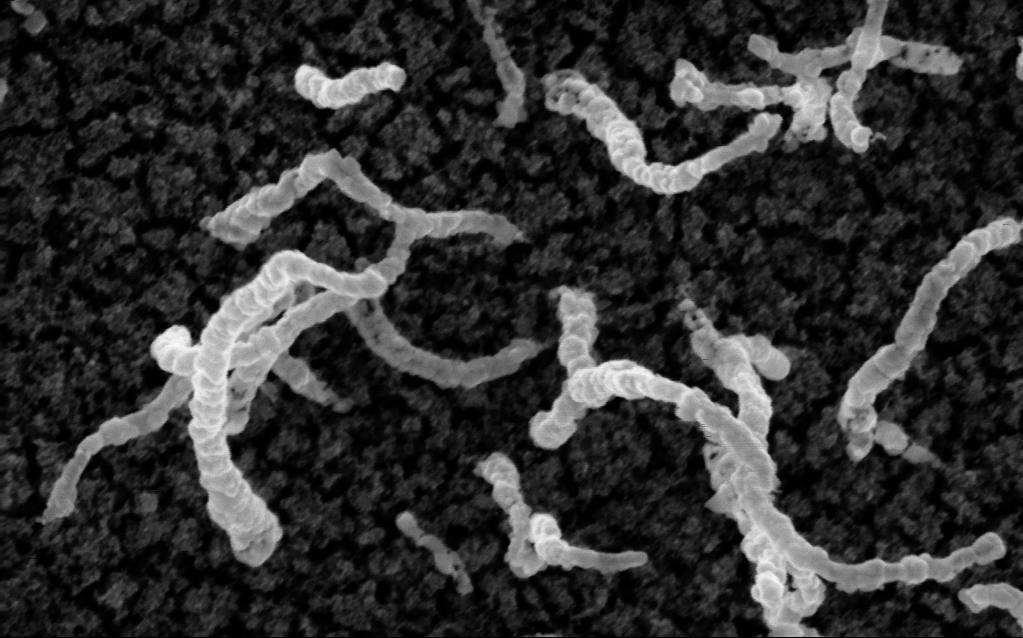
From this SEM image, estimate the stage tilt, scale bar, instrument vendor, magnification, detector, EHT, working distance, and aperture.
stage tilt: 0°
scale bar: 100 nm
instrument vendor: Zeiss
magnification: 200 K X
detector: InLens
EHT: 5 kV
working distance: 2.1 mm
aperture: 30 µm